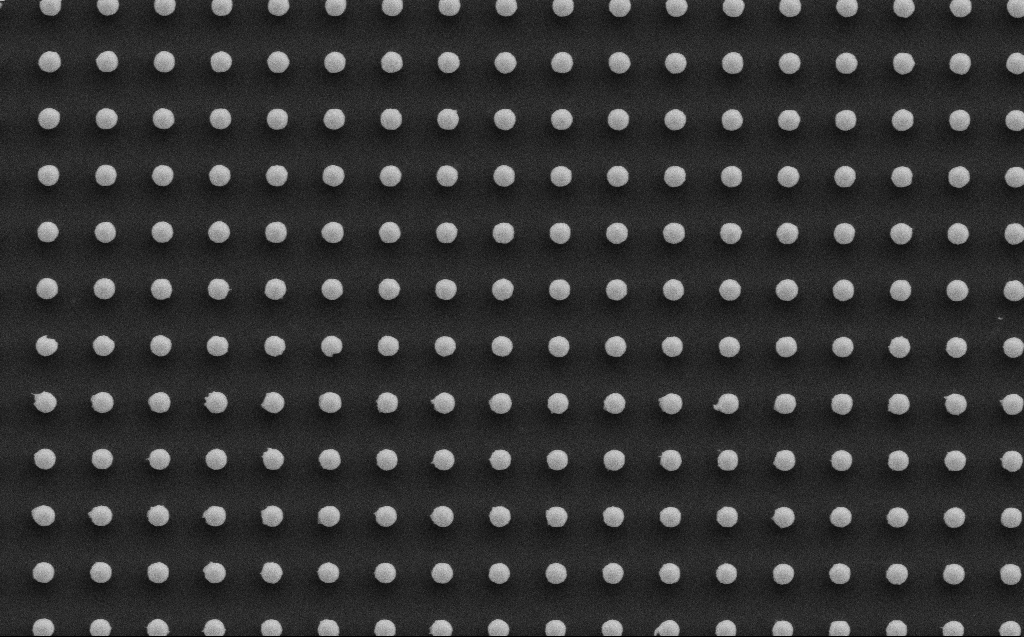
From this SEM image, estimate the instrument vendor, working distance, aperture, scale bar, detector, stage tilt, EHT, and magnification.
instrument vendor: Zeiss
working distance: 6 mm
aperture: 30 µm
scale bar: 2000 nm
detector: SE2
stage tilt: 0°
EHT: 5 kV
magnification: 20 K X